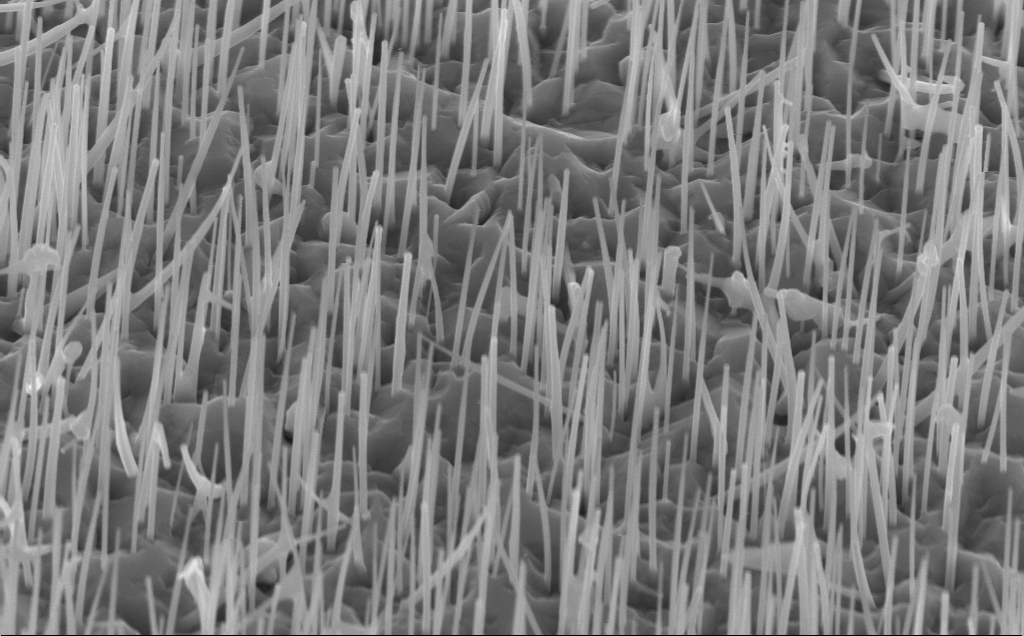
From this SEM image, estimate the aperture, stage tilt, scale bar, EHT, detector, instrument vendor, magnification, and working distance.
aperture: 30 µm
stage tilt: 45°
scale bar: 1000 nm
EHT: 10 kV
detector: InLens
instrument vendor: Zeiss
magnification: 50.98 K X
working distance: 5 mm